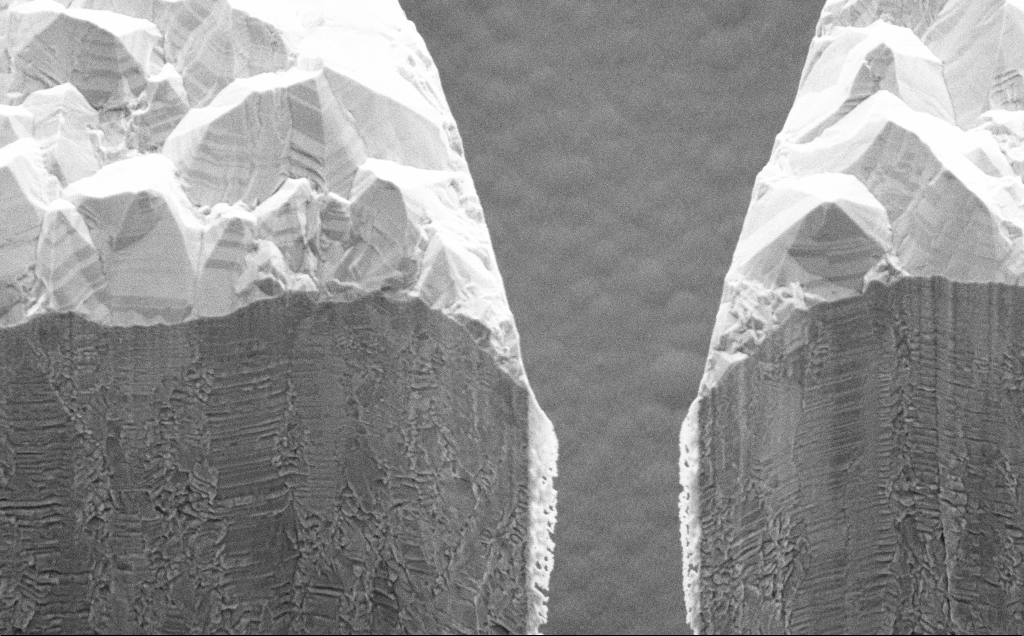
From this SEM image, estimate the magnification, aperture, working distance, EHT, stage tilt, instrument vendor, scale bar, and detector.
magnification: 19.32 K X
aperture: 30 µm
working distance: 10 mm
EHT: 5 kV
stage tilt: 45°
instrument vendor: Zeiss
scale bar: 2000 nm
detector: SE2